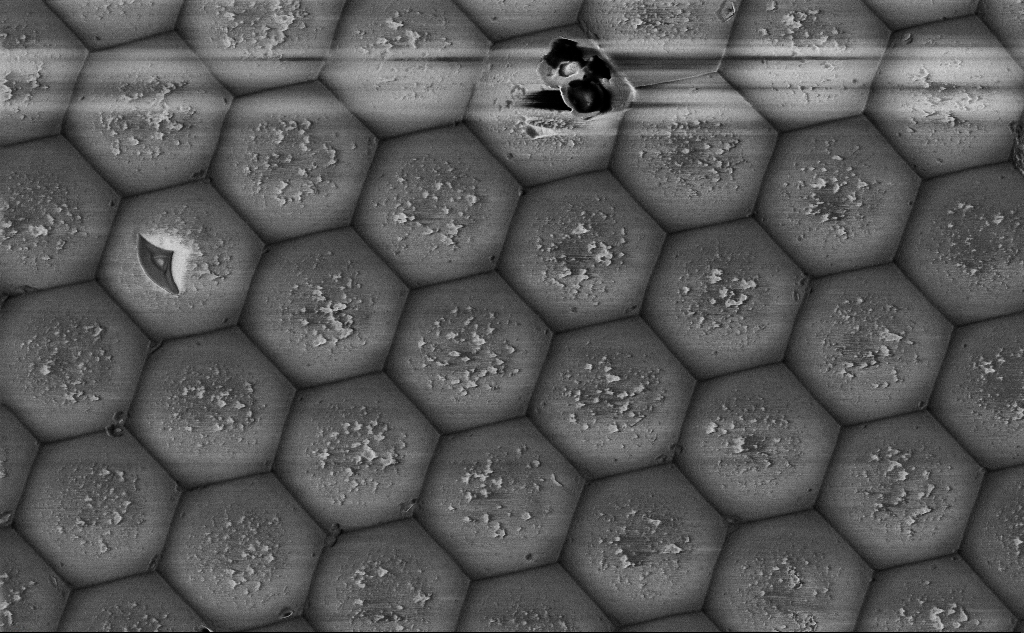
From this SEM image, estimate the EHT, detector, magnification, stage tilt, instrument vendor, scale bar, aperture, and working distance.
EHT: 3 kV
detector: InLens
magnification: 18.37 K X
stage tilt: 0°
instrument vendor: Zeiss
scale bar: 2000 nm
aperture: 30 µm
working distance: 6.6 mm